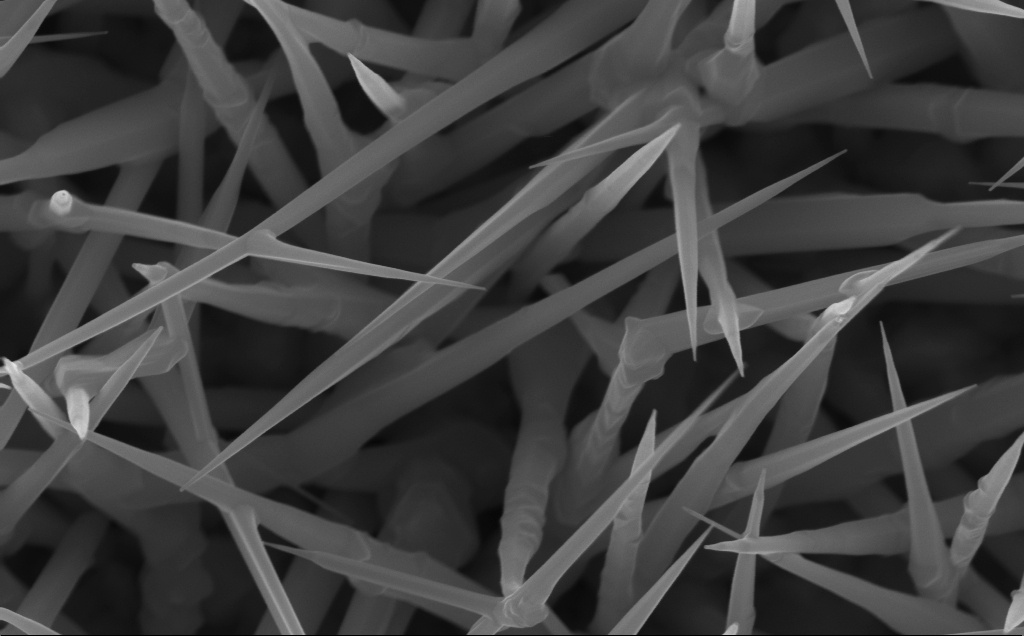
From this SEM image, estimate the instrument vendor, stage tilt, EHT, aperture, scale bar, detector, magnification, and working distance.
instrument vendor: Zeiss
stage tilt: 0°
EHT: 10 kV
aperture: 30 µm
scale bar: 200 nm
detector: InLens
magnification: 80 K X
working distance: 4 mm